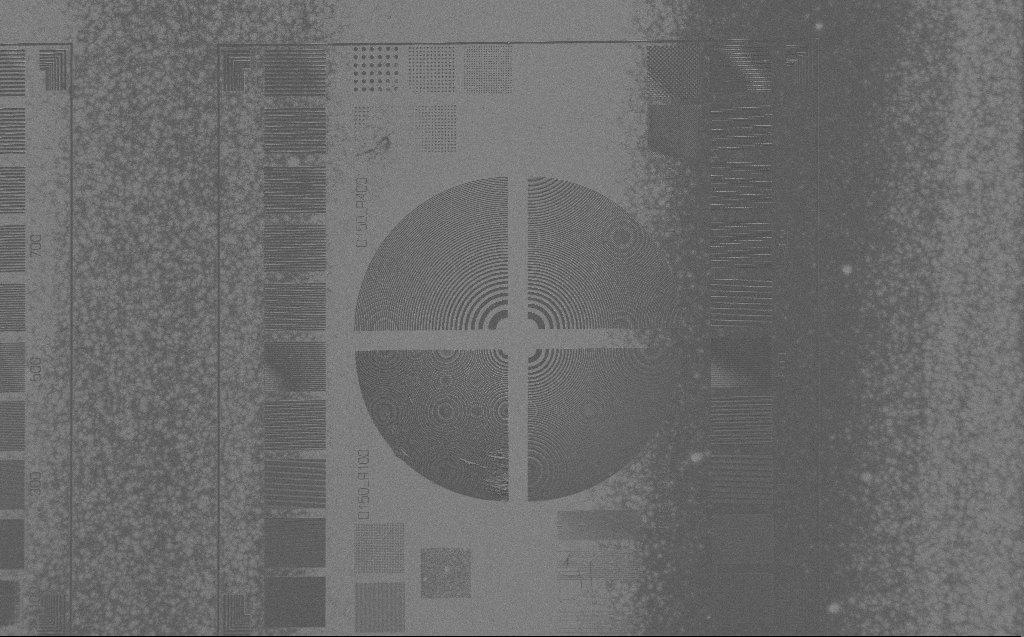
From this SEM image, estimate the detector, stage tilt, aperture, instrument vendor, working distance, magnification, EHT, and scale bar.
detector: SE2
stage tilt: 0°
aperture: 30 µm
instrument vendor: Zeiss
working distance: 5 mm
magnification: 0.747 K X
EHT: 1.2 kV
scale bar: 20000 nm